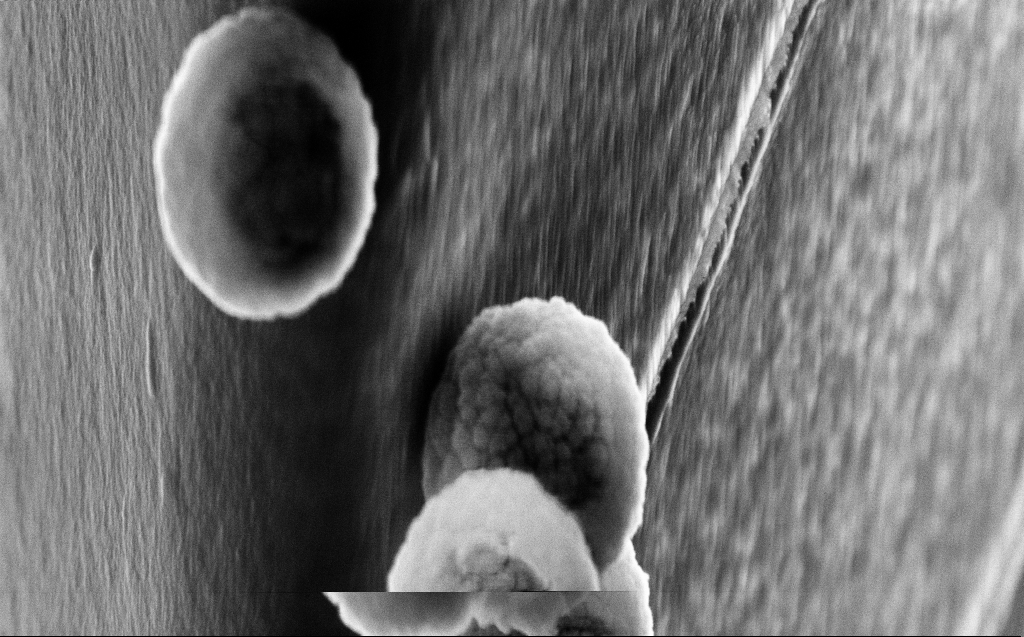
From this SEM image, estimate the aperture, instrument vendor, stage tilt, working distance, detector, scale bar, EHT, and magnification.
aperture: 30 µm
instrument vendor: Zeiss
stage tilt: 45°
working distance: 5 mm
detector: InLens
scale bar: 1000 nm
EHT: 10 kV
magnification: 54.8 K X